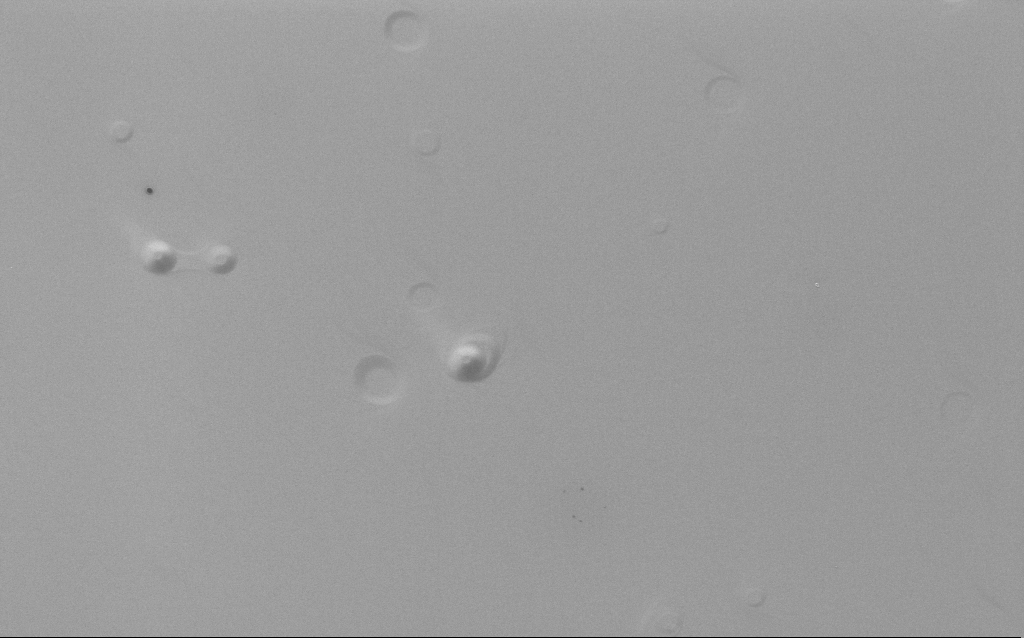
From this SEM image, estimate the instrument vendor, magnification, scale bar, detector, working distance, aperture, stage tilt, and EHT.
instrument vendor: Zeiss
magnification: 10.46 K X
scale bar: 2000 nm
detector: InLens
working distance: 4 mm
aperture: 30 µm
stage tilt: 20°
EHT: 5 kV